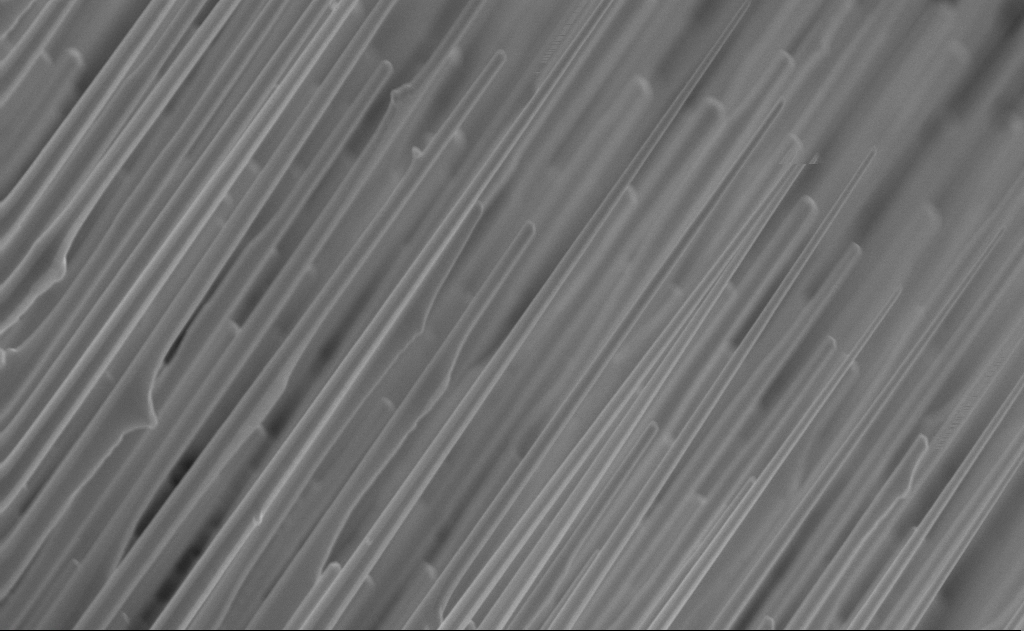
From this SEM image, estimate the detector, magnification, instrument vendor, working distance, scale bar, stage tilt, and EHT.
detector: InLens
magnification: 40 K X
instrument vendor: Zeiss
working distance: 6 mm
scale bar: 1000 nm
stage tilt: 0°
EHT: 10 kV